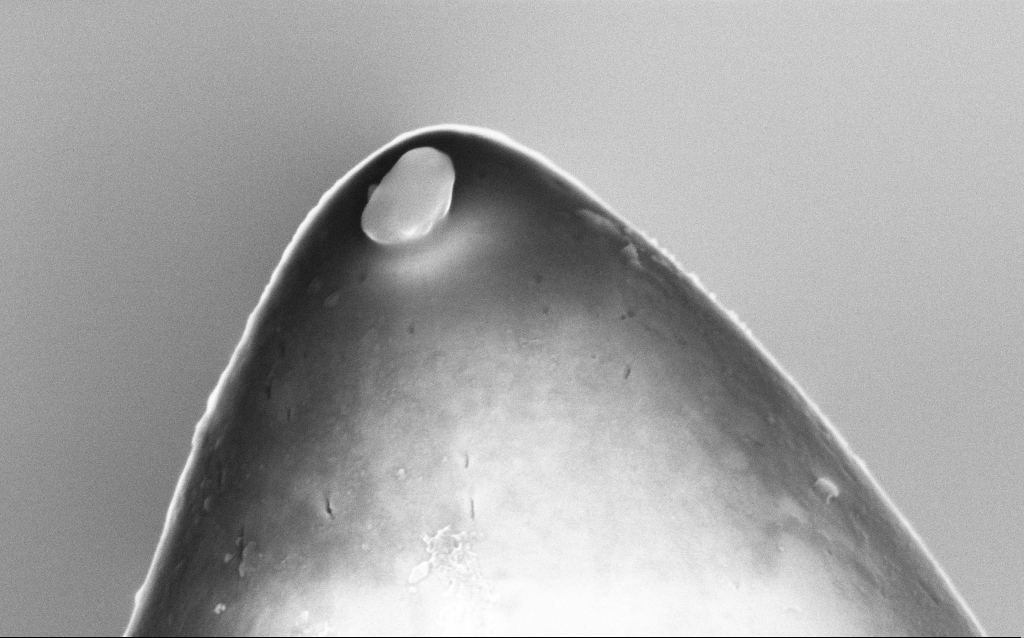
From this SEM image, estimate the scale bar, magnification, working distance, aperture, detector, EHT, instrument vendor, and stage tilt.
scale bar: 100 nm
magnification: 122.72 K X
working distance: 2.9 mm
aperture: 30 µm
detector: InLens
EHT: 5 kV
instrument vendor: Zeiss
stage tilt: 0°